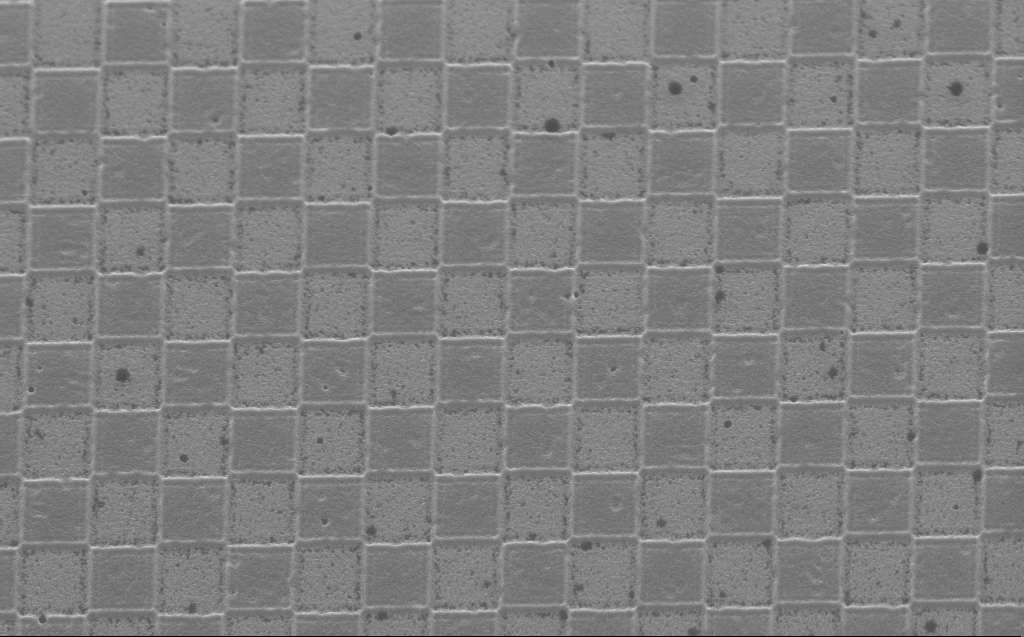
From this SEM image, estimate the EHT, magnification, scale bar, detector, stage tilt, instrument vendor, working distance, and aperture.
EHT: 5 kV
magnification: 25.5 K X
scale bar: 1000 nm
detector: InLens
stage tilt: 30°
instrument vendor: Zeiss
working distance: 4 mm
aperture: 30 µm